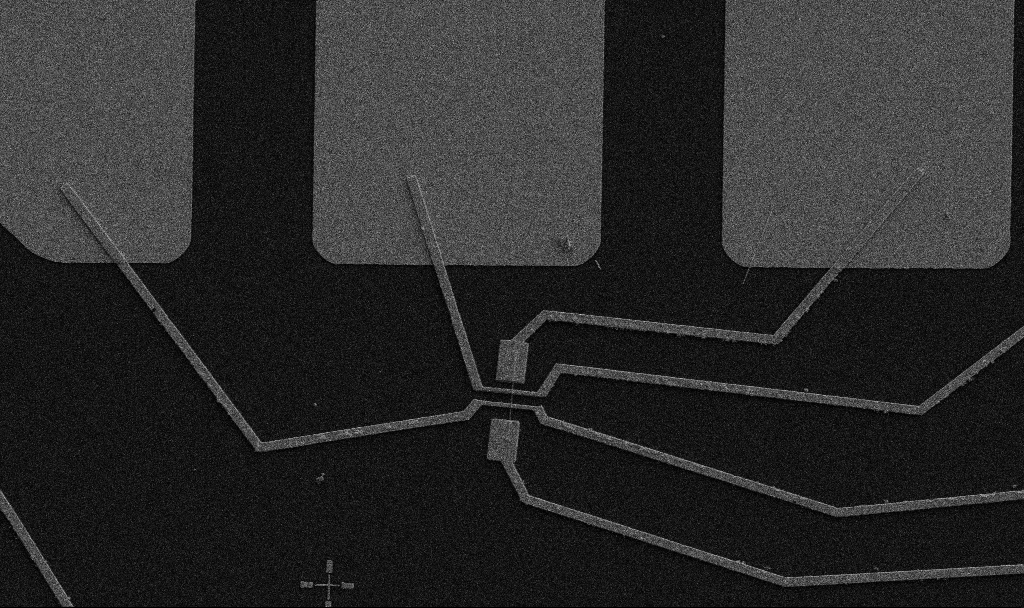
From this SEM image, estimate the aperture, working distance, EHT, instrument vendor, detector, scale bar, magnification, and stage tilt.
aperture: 30 µm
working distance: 10.7 mm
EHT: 5 kV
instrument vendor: Zeiss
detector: SE2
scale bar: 10000 nm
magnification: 5 K X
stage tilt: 0°